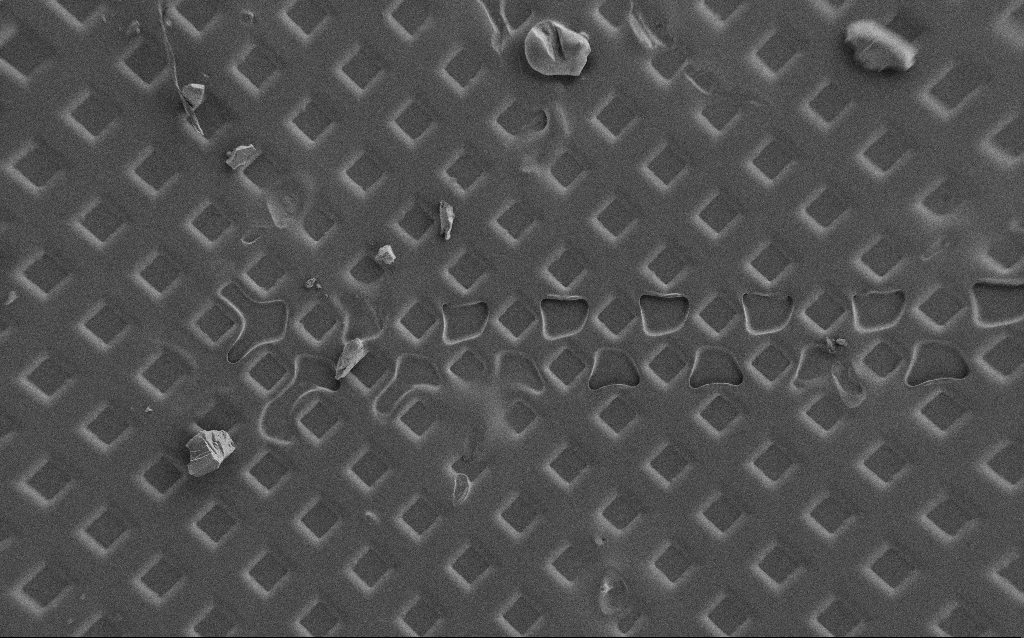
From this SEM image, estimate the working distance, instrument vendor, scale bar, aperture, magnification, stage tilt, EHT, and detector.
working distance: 8 mm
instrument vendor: Zeiss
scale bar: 100000 nm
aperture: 30 µm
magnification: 0.182 K X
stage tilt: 0°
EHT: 1.5 kV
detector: SE2